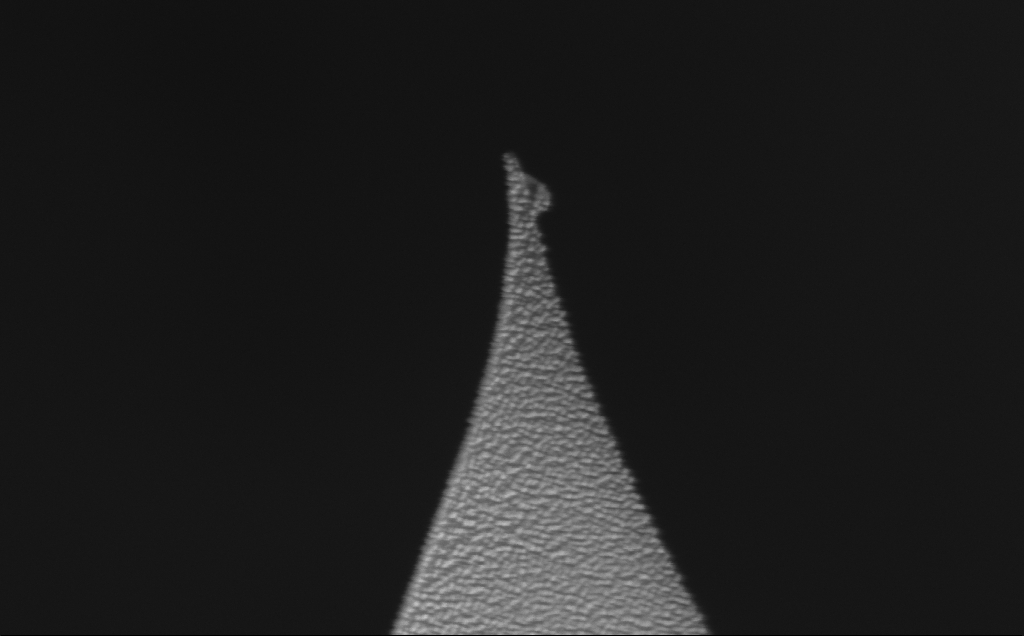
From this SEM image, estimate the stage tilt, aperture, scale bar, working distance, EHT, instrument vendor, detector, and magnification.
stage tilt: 52.7°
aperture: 30 µm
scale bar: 100 nm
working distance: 8 mm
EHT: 10 kV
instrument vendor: Zeiss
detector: InLens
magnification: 233.28 K X